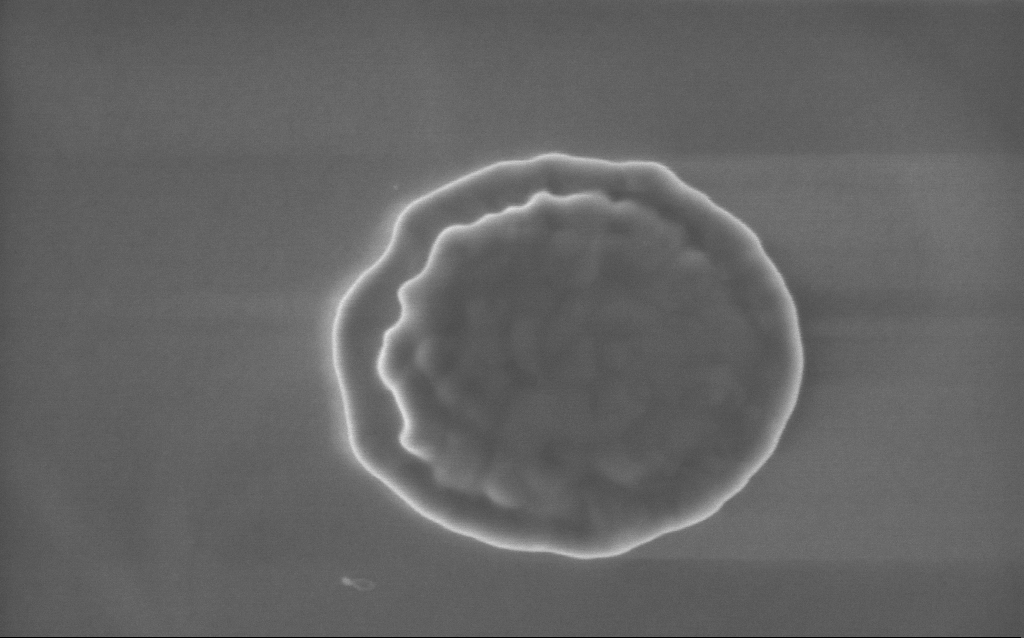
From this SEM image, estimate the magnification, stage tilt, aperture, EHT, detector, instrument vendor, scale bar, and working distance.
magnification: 89 K X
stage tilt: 0°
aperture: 30 µm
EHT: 5 kV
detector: InLens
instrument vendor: Zeiss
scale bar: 200 nm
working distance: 3 mm